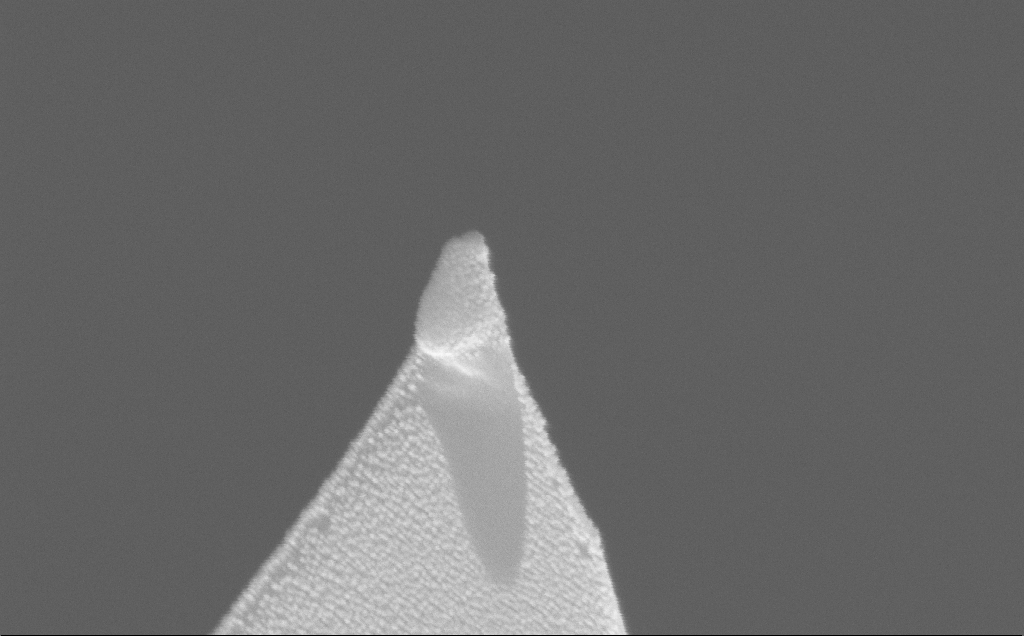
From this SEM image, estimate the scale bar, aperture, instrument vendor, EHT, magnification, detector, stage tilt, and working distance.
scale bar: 100 nm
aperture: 30 µm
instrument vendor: Zeiss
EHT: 10 kV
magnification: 195.84 K X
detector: InLens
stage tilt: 52.7°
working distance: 14 mm